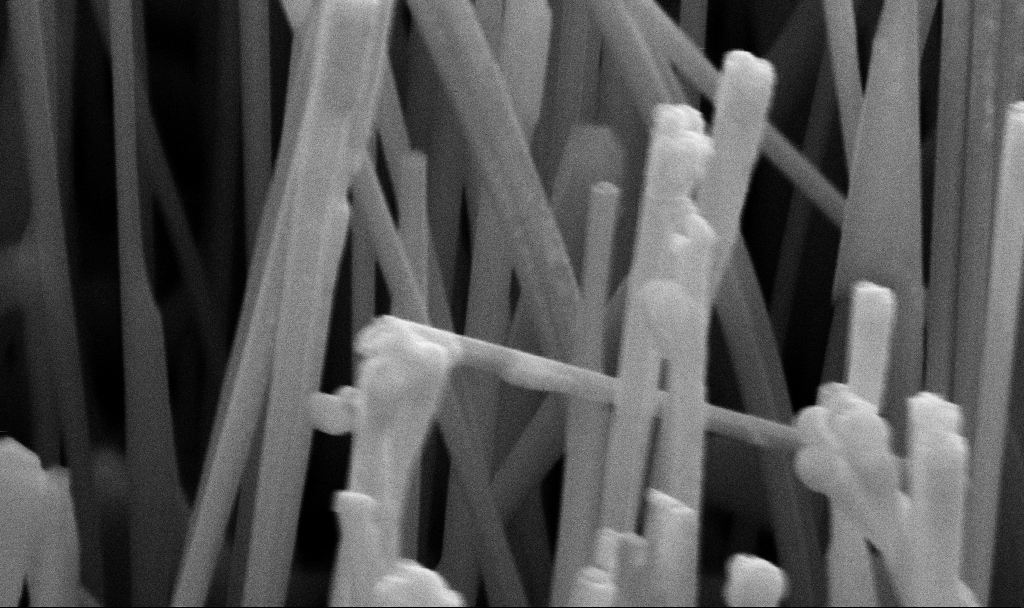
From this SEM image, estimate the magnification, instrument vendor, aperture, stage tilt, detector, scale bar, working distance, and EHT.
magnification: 99.6 K X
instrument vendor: Zeiss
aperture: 30 µm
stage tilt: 45°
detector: SE2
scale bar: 200 nm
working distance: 11.2 mm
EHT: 10 kV